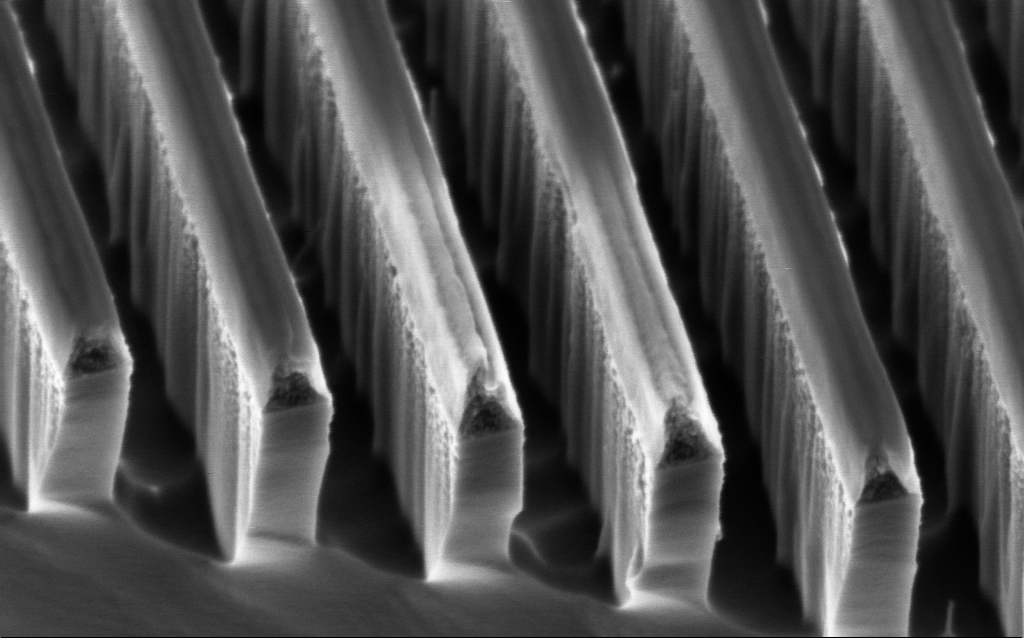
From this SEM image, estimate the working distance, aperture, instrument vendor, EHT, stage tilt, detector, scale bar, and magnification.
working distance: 3.6 mm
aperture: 30 µm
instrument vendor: Zeiss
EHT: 2 kV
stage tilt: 45°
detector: InLens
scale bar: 200 nm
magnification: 129.89 K X